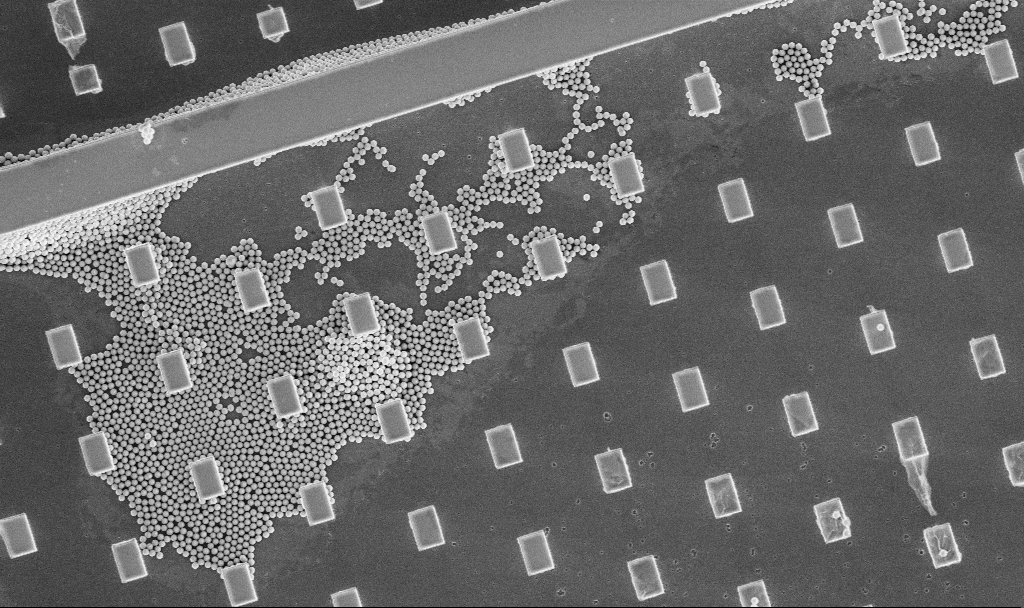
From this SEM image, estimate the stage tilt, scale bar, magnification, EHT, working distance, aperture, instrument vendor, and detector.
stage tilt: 0°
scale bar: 10000 nm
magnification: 3.43 K X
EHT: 5 kV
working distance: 4.9 mm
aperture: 30 µm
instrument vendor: Zeiss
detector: InLens